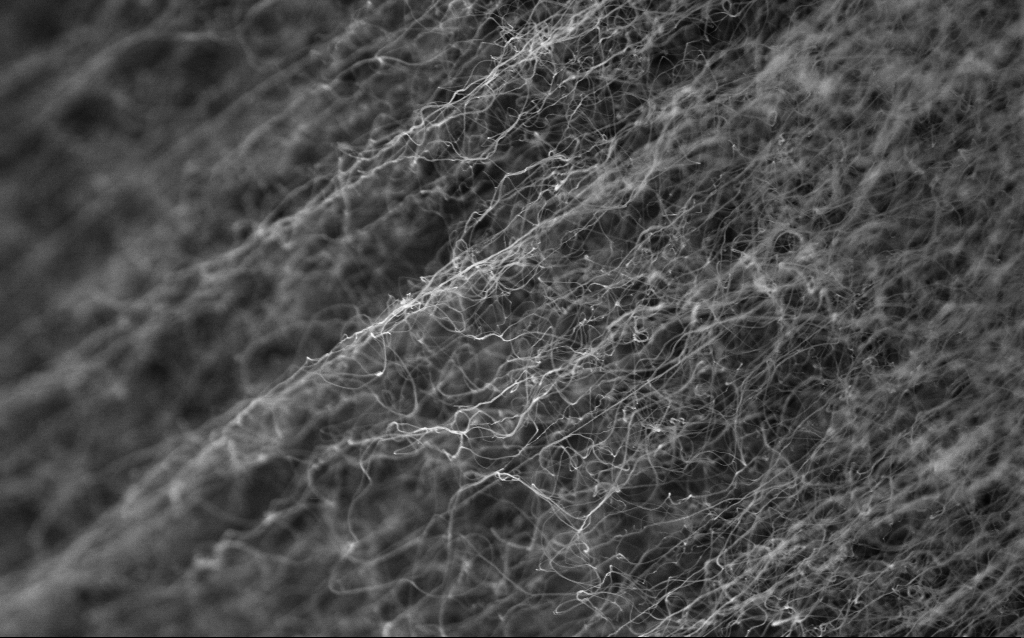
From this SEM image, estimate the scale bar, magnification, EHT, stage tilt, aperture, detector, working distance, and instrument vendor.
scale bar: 1000 nm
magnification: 44.7 K X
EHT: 5 kV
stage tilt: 0°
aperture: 30 µm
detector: InLens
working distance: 2.2 mm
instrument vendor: Zeiss